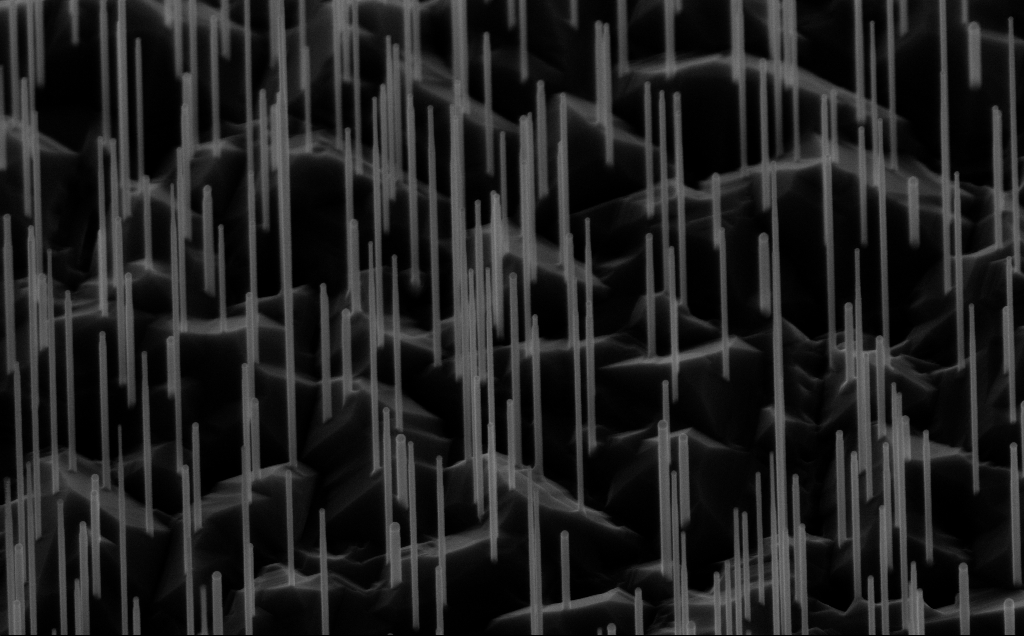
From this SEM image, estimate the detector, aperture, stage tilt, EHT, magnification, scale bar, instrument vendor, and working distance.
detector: InLens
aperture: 30 µm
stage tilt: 45°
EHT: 10 kV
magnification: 40 K X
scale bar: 1000 nm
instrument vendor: Zeiss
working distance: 6 mm